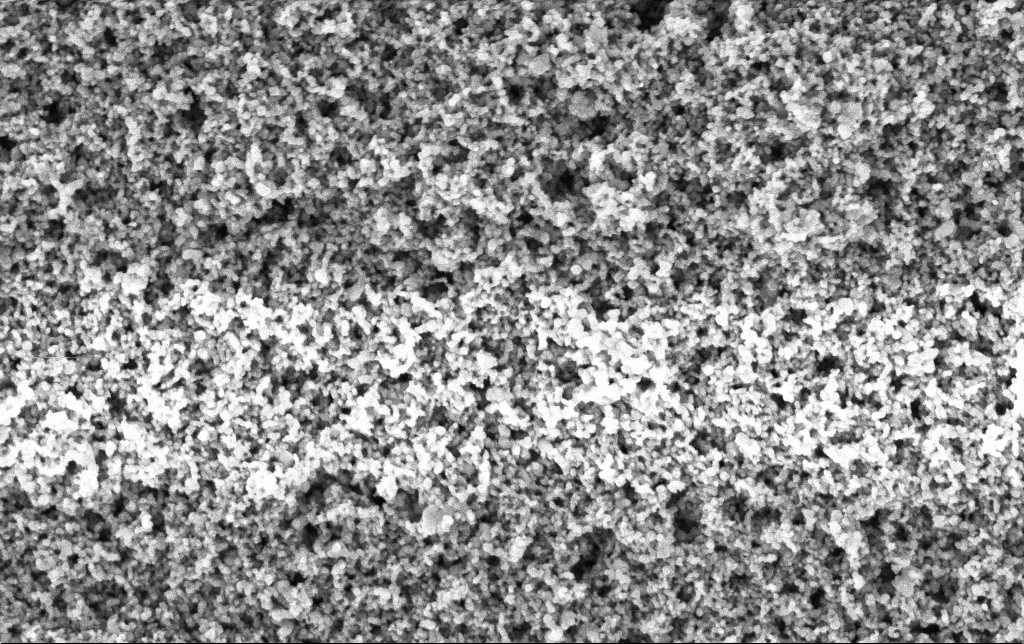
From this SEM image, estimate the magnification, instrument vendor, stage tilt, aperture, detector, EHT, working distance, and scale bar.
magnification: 80 K X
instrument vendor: Zeiss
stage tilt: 0°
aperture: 30 µm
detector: InLens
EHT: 3 kV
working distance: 2.8 mm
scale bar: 200 nm